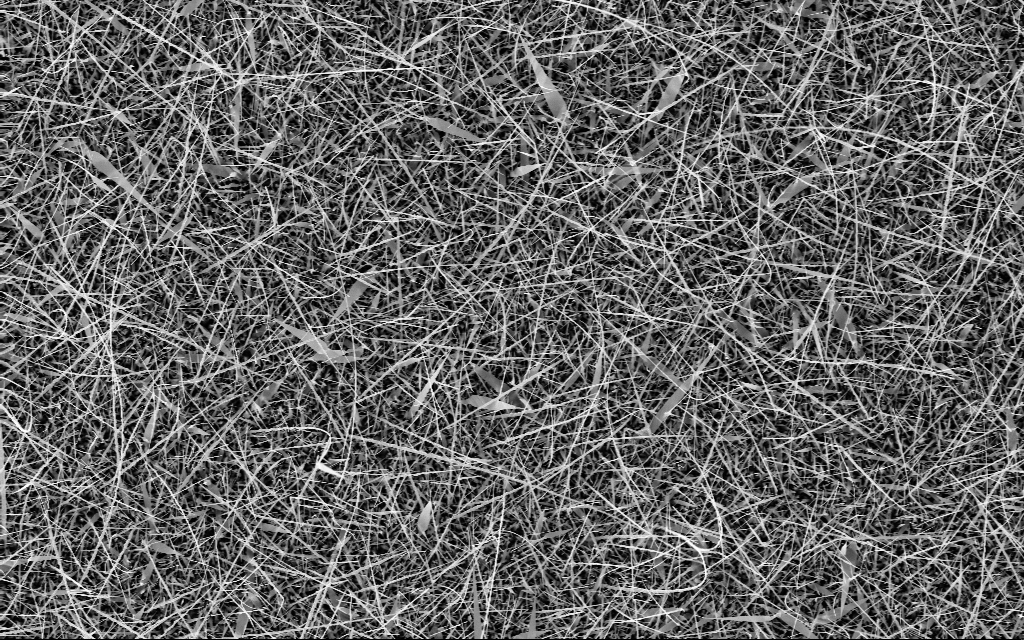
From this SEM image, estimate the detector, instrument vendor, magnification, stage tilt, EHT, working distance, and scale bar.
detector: InLens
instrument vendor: Zeiss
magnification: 5 K X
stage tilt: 0°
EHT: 10 kV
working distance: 6 mm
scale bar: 10000 nm